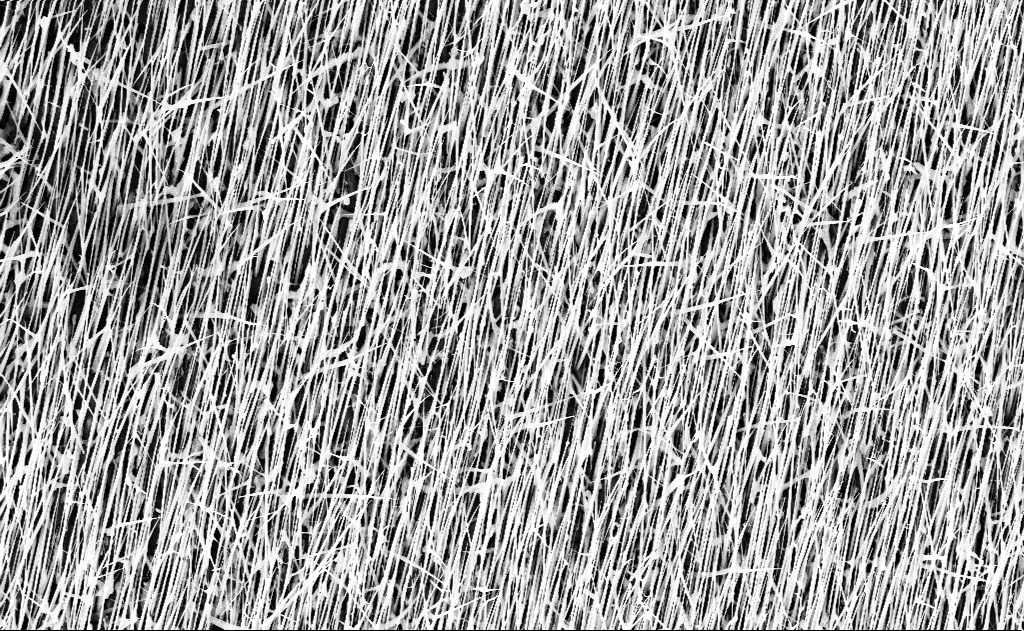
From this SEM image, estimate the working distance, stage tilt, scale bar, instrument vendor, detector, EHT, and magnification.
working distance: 16 mm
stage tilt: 0°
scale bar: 2000 nm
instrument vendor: Zeiss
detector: InLens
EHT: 10 kV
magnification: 10 K X